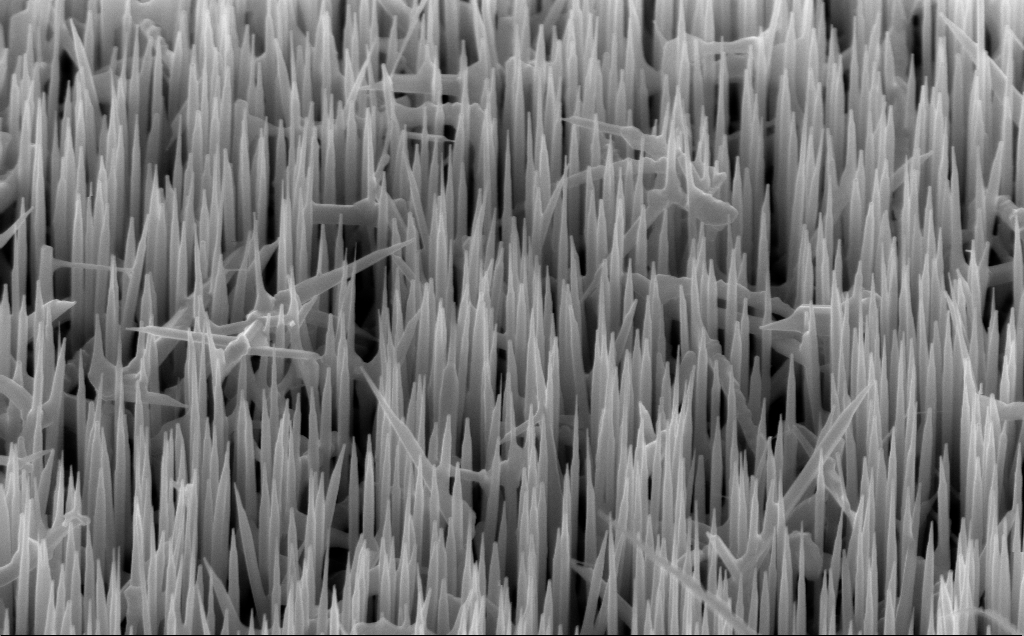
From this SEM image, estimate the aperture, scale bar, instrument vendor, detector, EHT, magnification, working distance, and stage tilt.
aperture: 30 µm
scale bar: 1000 nm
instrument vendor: Zeiss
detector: InLens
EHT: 10 kV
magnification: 40 K X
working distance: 6 mm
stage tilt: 45°